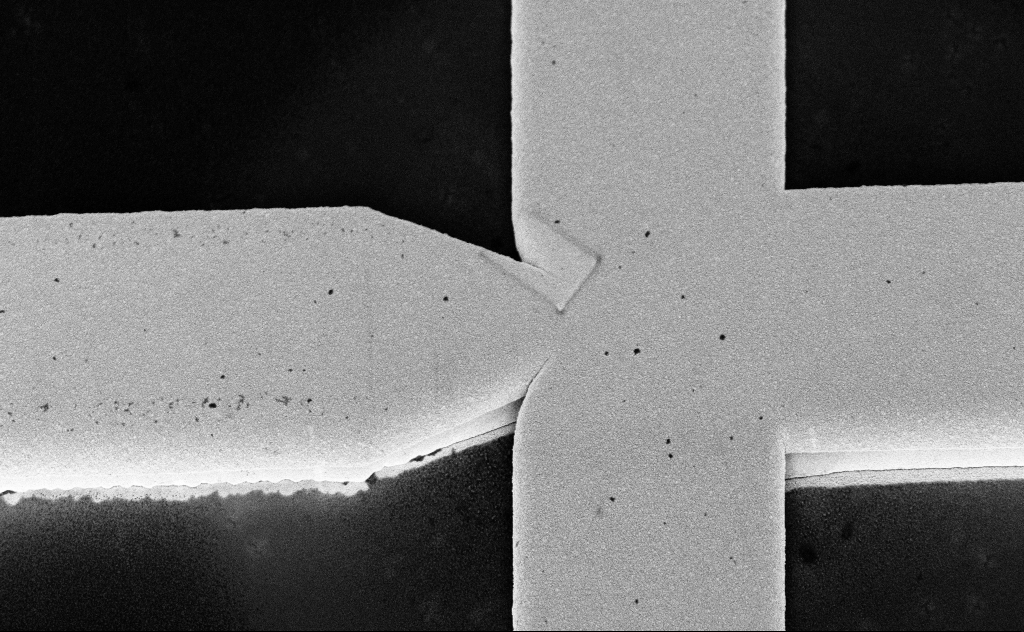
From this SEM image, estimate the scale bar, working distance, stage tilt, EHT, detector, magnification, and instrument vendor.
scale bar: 2000 nm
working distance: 12 mm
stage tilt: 45°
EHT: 3 kV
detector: InLens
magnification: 7.97 K X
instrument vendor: Zeiss